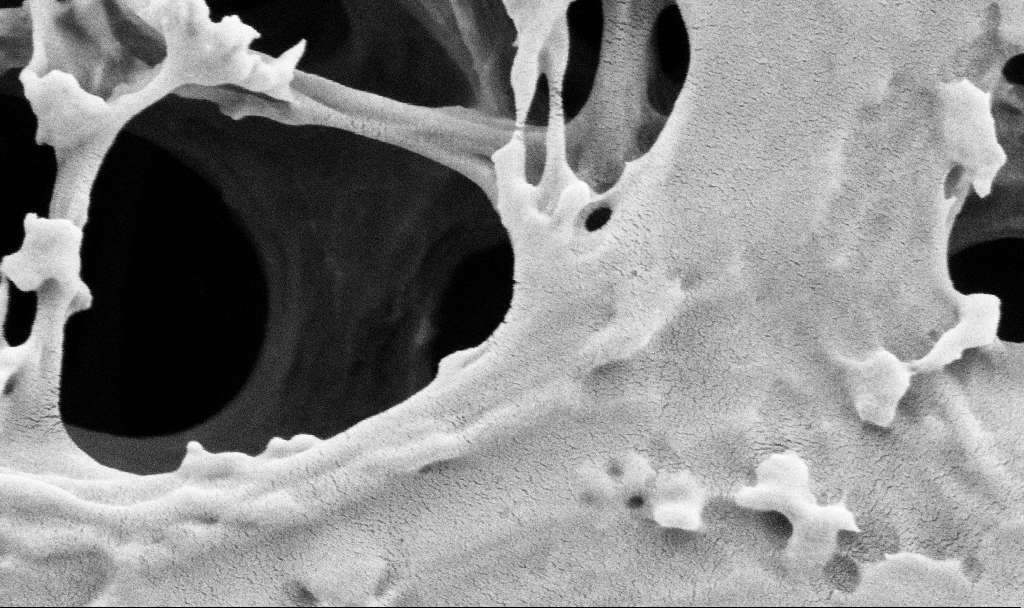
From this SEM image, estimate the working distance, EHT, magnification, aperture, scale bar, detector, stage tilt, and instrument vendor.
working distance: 3.5 mm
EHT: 2 kV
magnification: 100 K X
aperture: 30 µm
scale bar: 200 nm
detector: SE2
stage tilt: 0°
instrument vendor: Zeiss